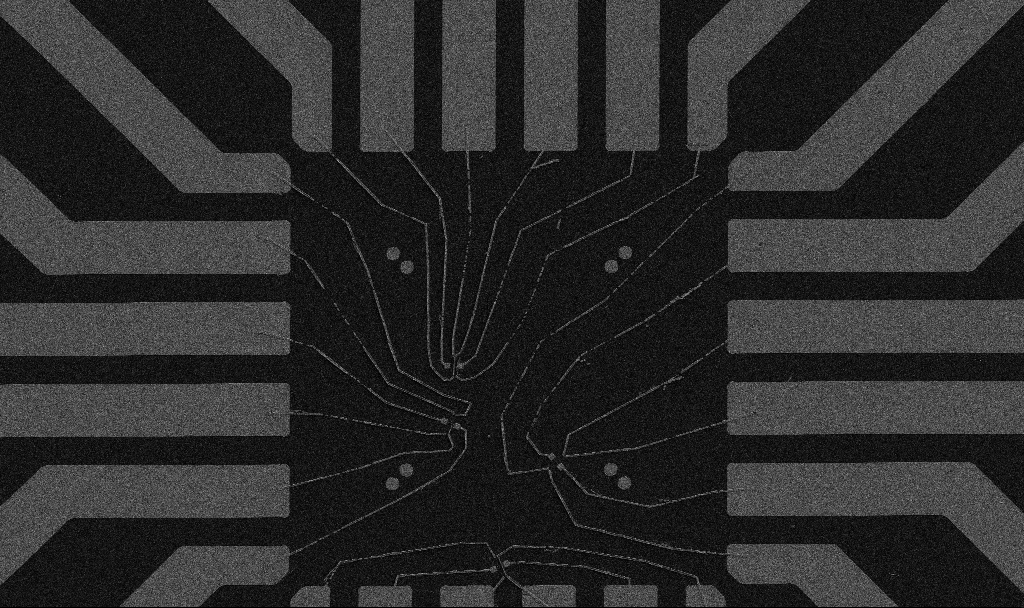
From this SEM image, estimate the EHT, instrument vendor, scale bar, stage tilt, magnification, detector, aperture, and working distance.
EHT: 5 kV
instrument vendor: Zeiss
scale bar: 20000 nm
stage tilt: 0°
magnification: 1 K X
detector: SE2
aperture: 30 µm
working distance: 10.7 mm